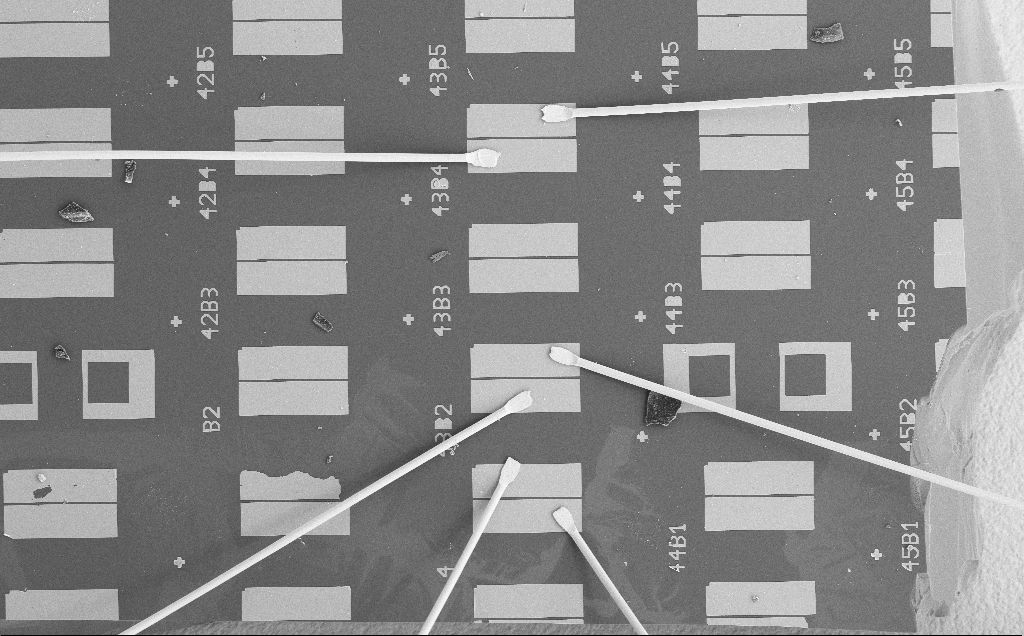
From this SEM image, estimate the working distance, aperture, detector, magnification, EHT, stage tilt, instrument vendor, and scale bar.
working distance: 11 mm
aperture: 30 µm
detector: SE2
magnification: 0.128 K X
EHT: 15 kV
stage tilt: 0°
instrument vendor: Zeiss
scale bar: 200000 nm